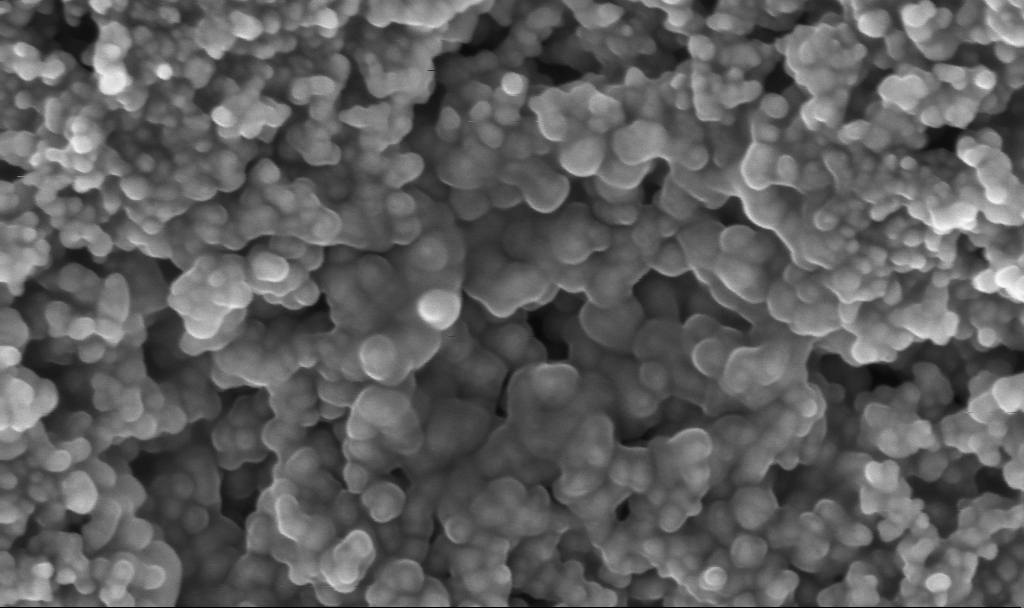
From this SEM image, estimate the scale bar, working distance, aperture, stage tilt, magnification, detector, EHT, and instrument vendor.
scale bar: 100 nm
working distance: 2.4 mm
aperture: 30 µm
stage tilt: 0°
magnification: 200 K X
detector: InLens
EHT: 3 kV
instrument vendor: Zeiss